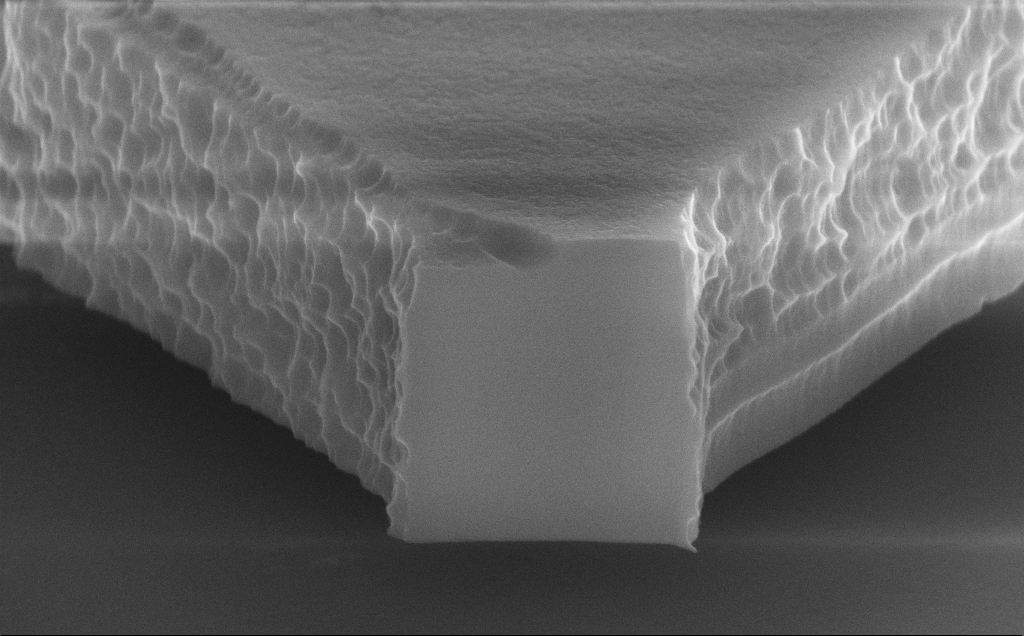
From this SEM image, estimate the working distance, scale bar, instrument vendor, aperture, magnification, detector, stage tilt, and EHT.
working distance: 9 mm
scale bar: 1000 nm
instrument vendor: Zeiss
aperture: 30 µm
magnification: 47.87 K X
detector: InLens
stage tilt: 70°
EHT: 10 kV